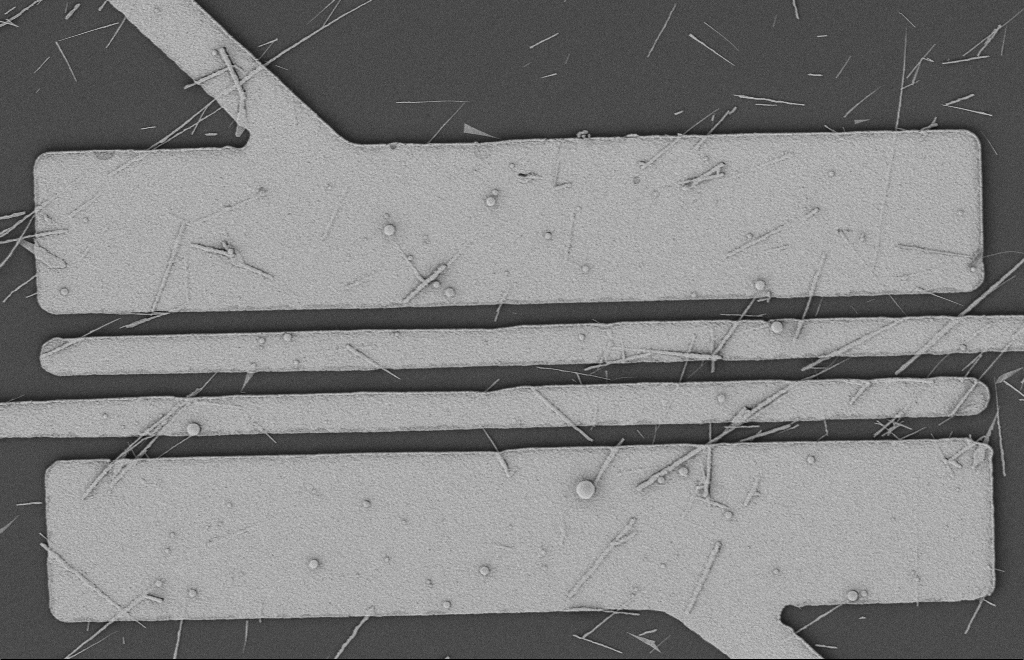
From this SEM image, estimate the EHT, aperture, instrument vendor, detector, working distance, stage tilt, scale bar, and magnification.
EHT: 2 kV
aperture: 20 µm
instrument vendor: Zeiss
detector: SE2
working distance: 12 mm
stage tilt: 0°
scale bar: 2000 nm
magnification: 5.65 K X